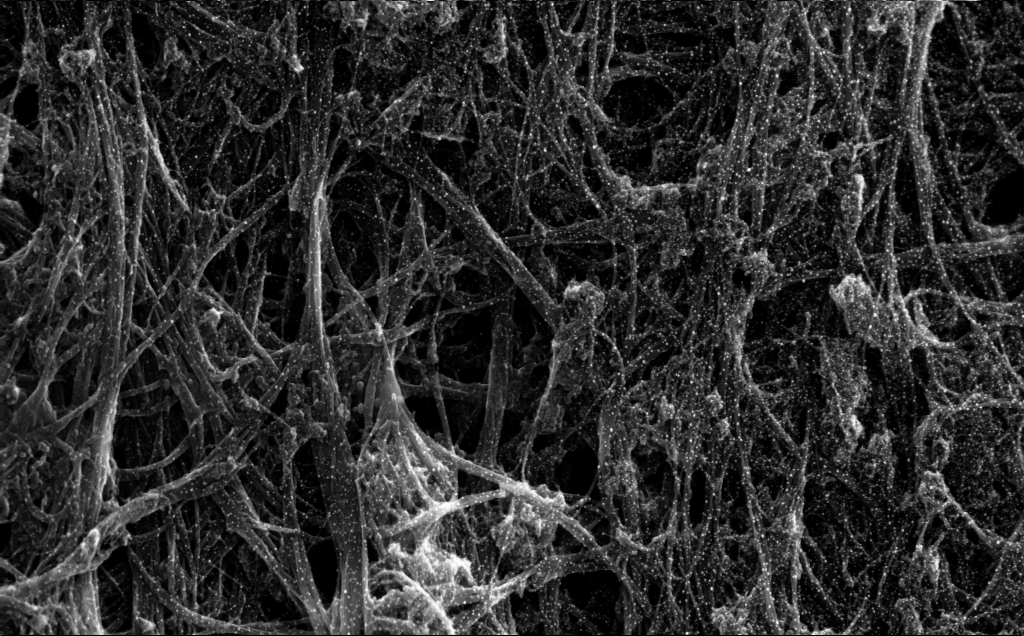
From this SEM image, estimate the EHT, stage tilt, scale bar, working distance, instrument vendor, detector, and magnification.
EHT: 5 kV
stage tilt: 0°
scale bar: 200 nm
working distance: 4 mm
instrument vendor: Zeiss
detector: InLens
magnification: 91.52 K X